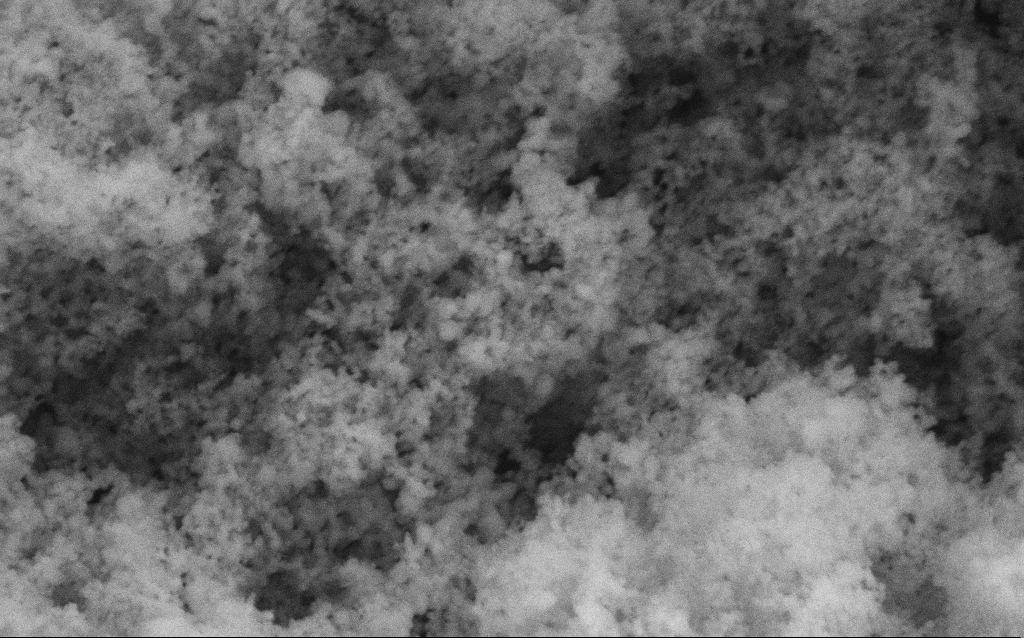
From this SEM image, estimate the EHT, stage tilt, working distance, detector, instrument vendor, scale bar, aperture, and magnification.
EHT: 5 kV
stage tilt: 0°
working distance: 4.1 mm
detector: SE2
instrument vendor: Zeiss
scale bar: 200 nm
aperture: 30 µm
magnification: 114.64 K X